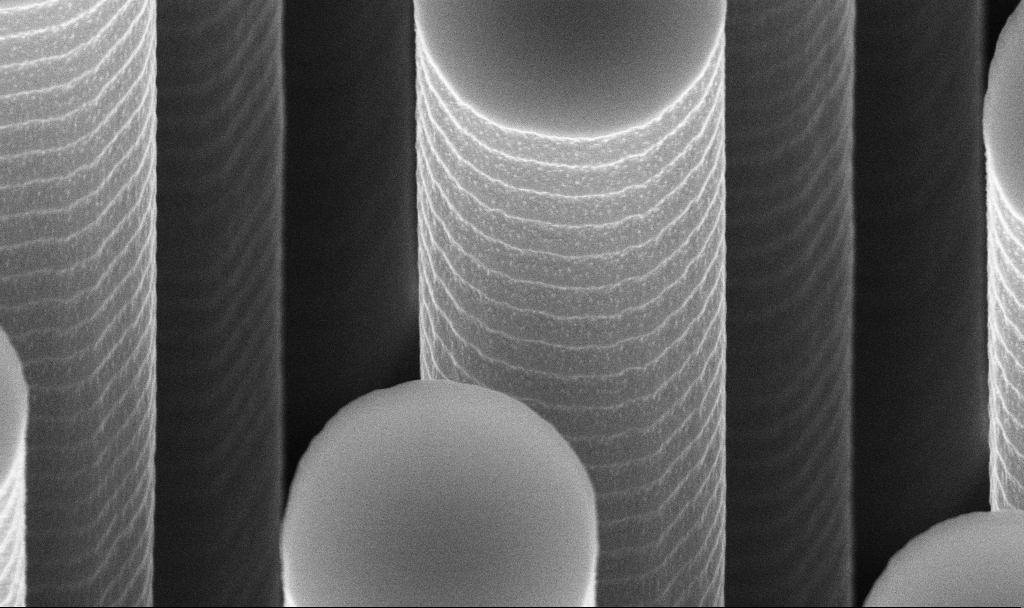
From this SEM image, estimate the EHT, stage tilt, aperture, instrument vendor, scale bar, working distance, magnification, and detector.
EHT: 10 kV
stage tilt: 45°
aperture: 30 µm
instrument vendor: Zeiss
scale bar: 1000 nm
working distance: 7.8 mm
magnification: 54.16 K X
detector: InLens